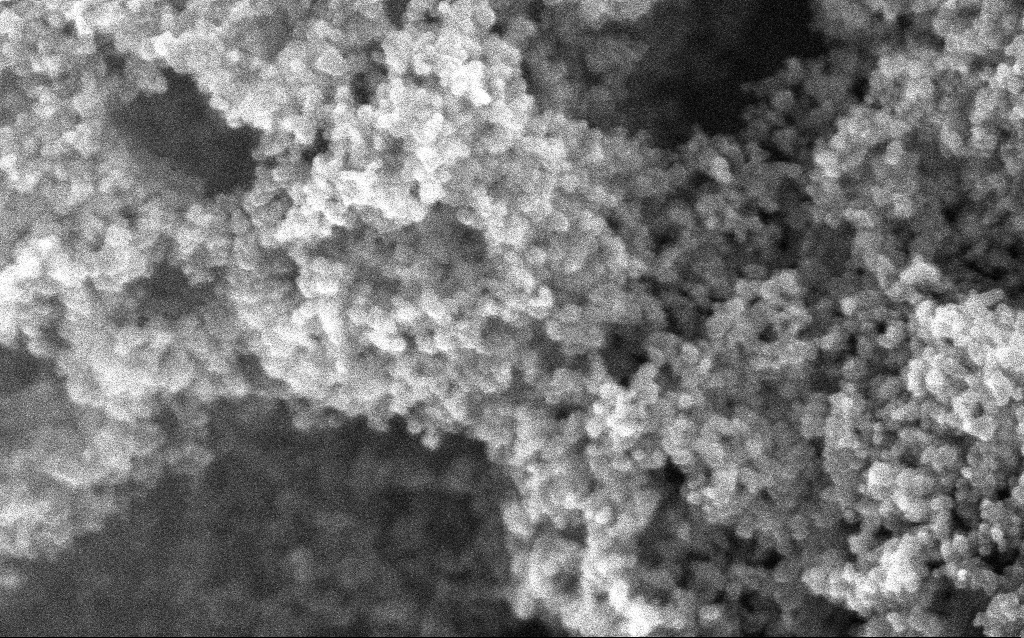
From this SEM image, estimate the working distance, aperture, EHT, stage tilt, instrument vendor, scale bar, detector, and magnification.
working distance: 2.4 mm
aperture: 30 µm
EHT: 10 kV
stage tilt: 0°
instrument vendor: Zeiss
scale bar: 100 nm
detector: InLens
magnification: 211.33 K X